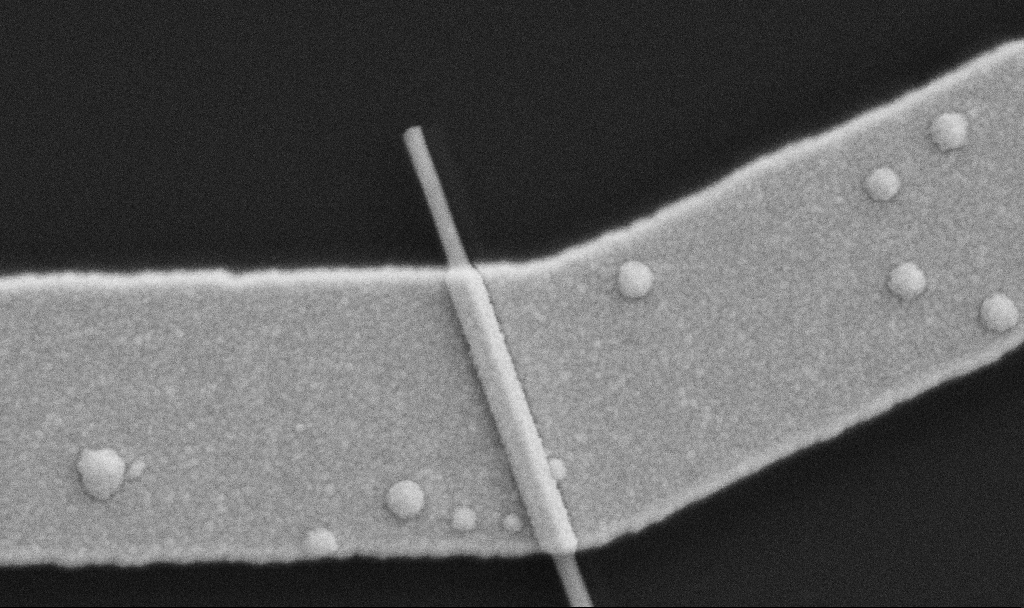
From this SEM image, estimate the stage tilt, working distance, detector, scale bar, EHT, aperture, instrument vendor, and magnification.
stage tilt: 0°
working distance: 10.7 mm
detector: SE2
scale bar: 200 nm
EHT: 5 kV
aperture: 30 µm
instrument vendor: Zeiss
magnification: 100 K X